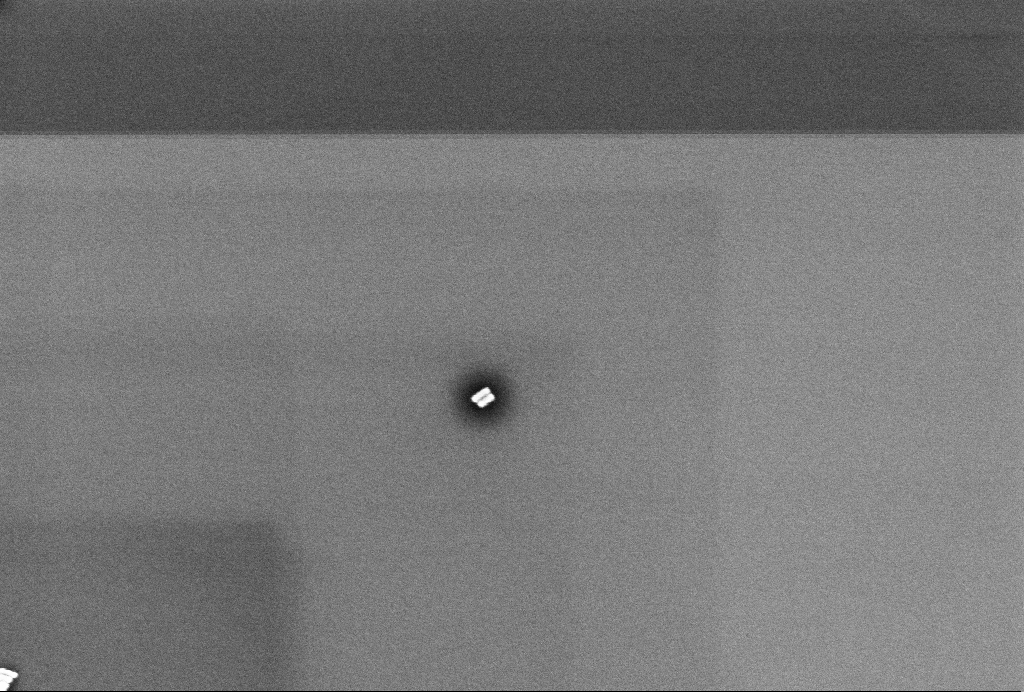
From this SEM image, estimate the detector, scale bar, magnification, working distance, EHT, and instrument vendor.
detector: InLens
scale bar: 200 nm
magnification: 92.4 K X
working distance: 3.3 mm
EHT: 2 kV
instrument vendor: Zeiss